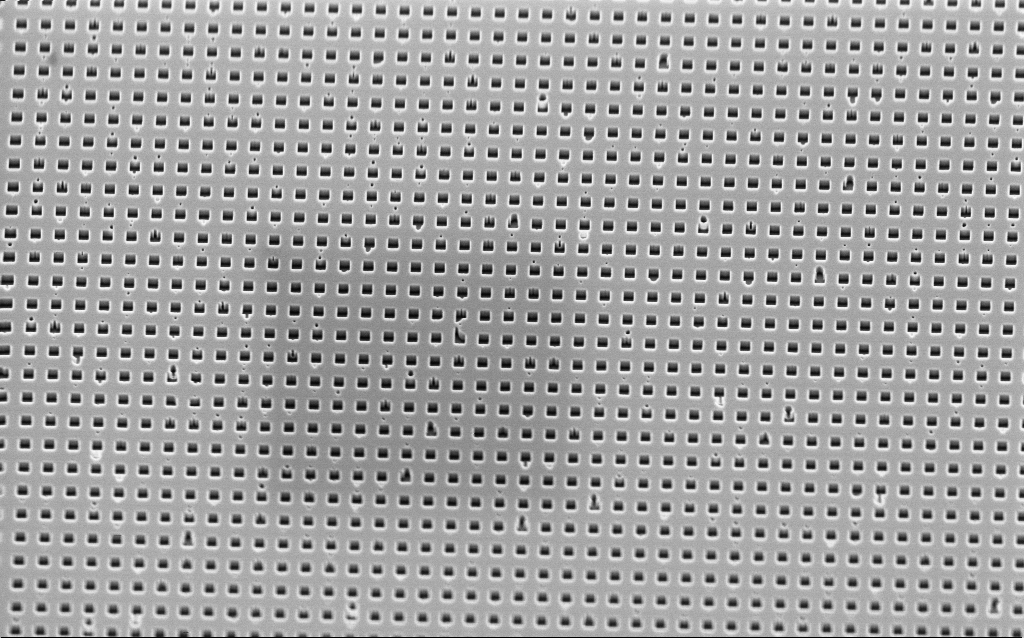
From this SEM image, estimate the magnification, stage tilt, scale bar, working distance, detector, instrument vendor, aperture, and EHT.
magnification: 17.58 K X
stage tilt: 45°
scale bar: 2000 nm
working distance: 2.7 mm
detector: InLens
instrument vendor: Zeiss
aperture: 30 µm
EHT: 2 kV